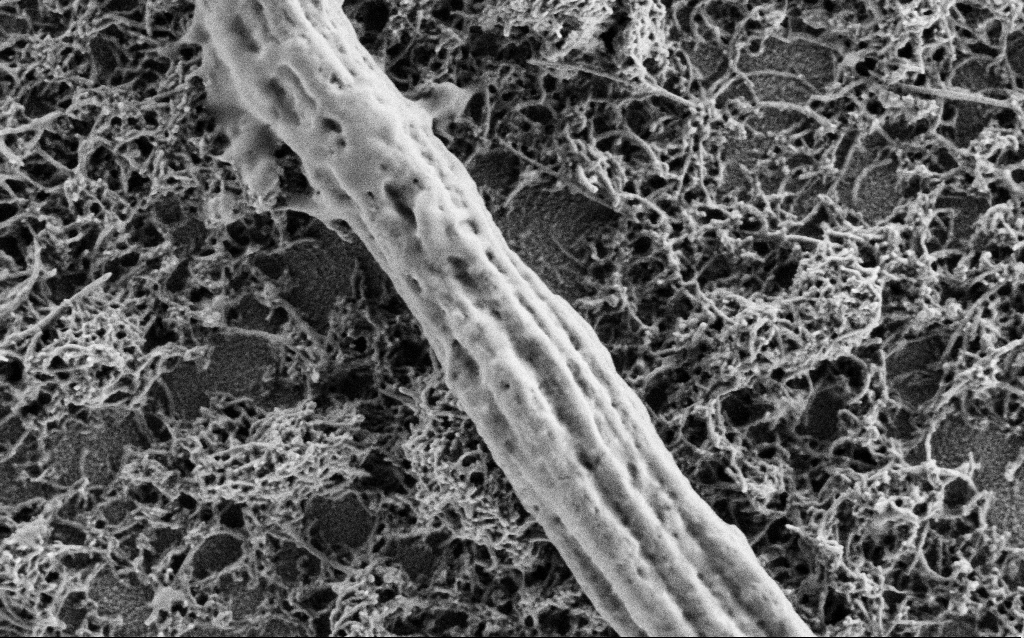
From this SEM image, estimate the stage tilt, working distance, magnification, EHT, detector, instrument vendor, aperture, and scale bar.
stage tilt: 0°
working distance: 4 mm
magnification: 50 K X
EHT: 1 kV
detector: SE2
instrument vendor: Zeiss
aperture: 30 µm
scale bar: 1000 nm